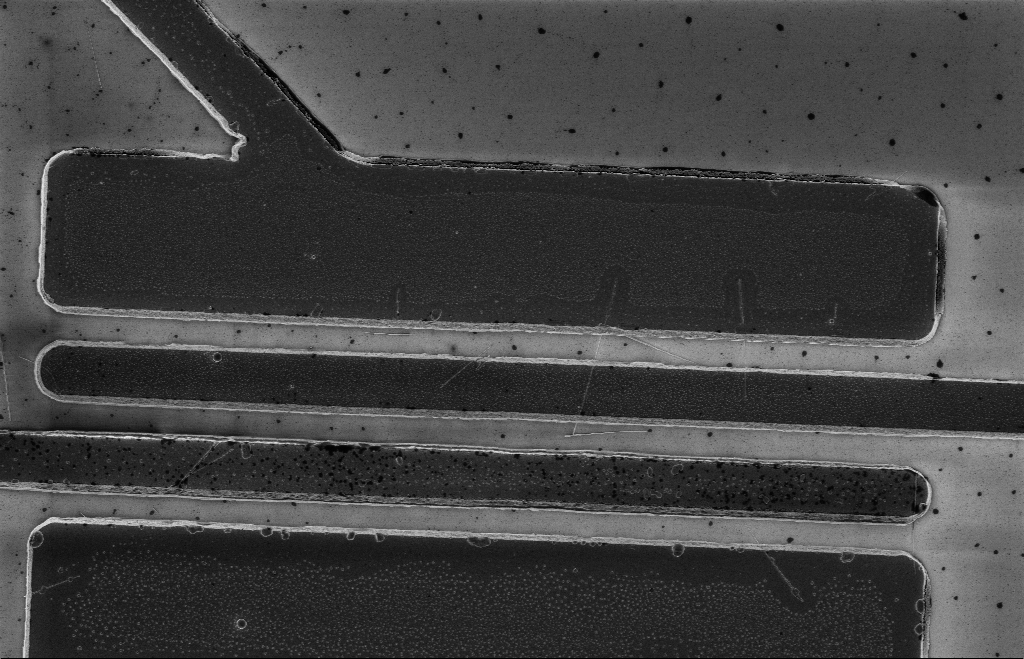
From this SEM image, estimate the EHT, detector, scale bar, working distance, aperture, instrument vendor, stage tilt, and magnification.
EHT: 5 kV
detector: InLens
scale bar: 2000 nm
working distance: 12 mm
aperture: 20 µm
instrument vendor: Zeiss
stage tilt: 0°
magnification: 5.43 K X